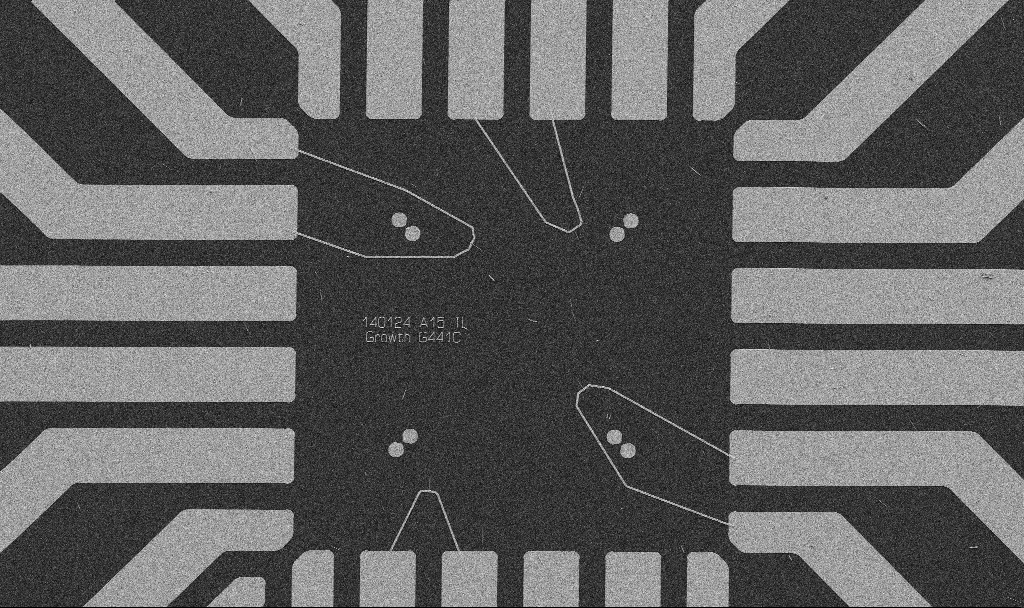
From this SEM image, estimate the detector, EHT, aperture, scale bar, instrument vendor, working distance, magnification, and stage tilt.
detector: SE2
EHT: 5 kV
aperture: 30 µm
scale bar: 20000 nm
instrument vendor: Zeiss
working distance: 10.7 mm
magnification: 1 K X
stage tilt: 0°